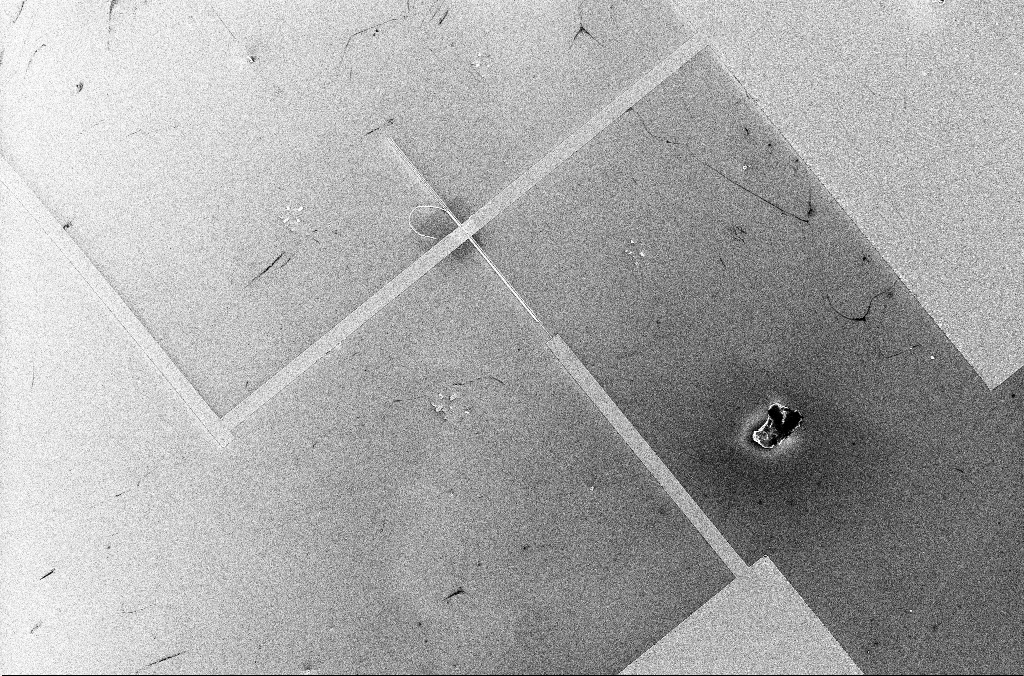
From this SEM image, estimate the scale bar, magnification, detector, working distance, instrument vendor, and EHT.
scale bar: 20000 nm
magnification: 1.13 K X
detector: InLens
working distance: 3.3 mm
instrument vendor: Zeiss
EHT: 5 kV